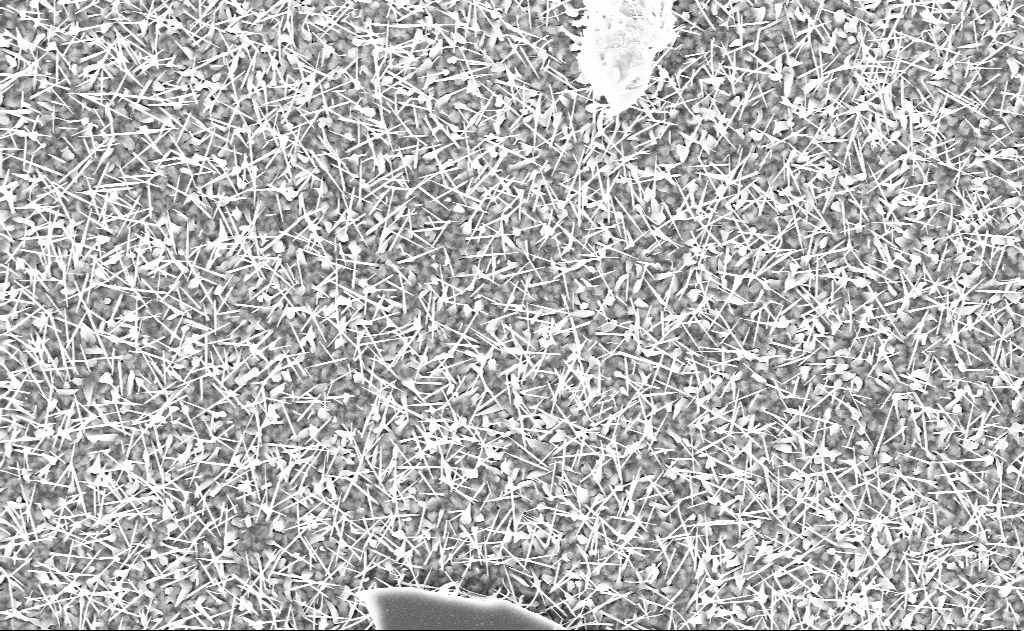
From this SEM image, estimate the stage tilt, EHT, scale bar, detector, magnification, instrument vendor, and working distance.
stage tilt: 0°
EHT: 10 kV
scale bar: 2000 nm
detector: InLens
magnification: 10 K X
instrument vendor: Zeiss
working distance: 9 mm